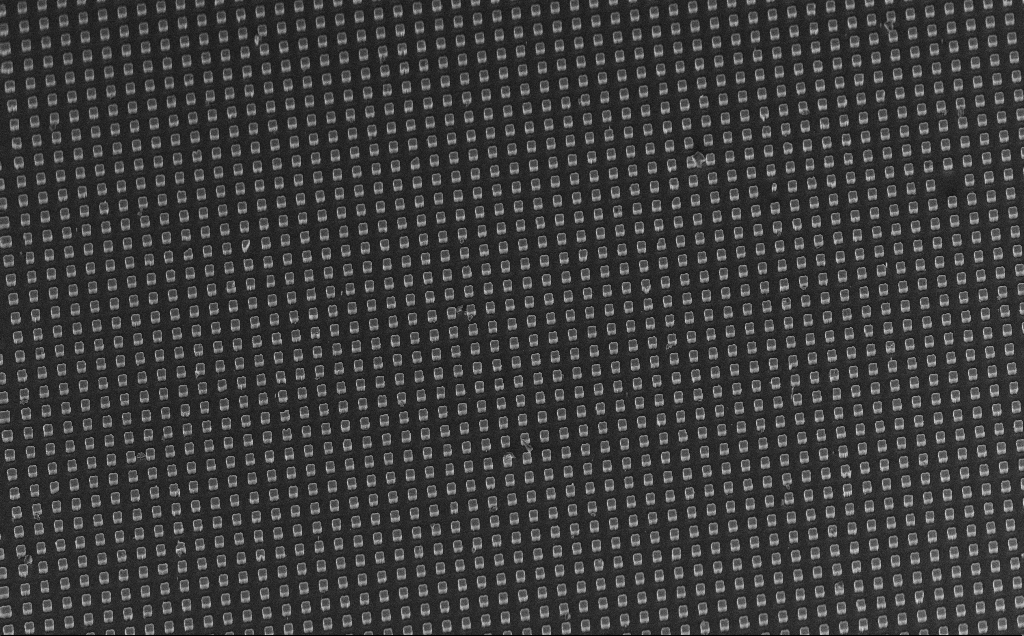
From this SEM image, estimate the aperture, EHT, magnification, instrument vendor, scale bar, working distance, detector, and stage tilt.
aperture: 30 µm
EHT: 10 kV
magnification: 0.718 K X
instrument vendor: Zeiss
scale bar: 100000 nm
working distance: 11 mm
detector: InLens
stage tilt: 45°